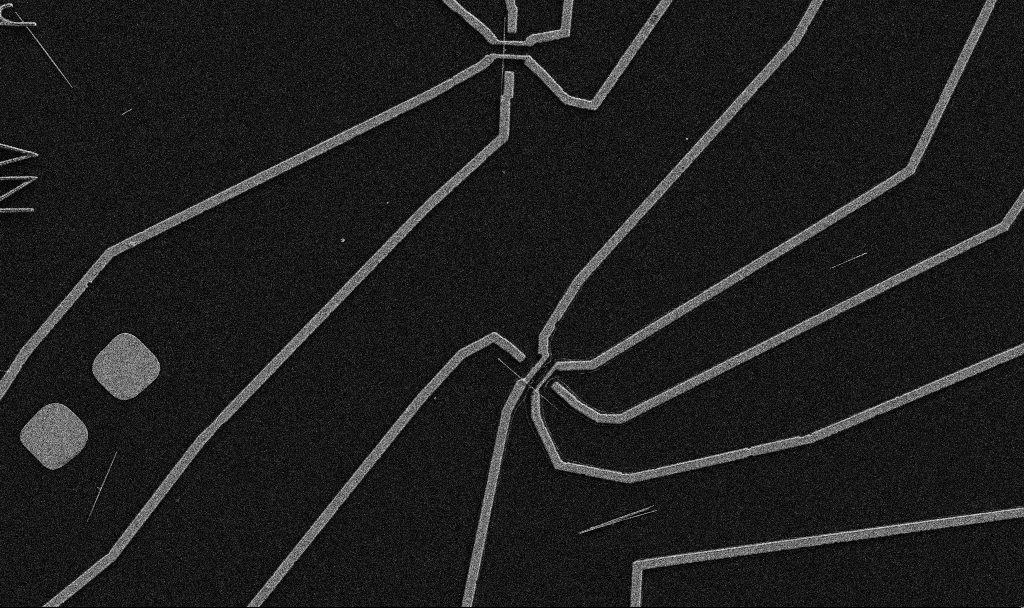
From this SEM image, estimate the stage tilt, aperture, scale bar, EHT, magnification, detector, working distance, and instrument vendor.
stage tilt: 0°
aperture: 30 µm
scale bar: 10000 nm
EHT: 5 kV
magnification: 5 K X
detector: SE2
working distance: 10.7 mm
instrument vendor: Zeiss